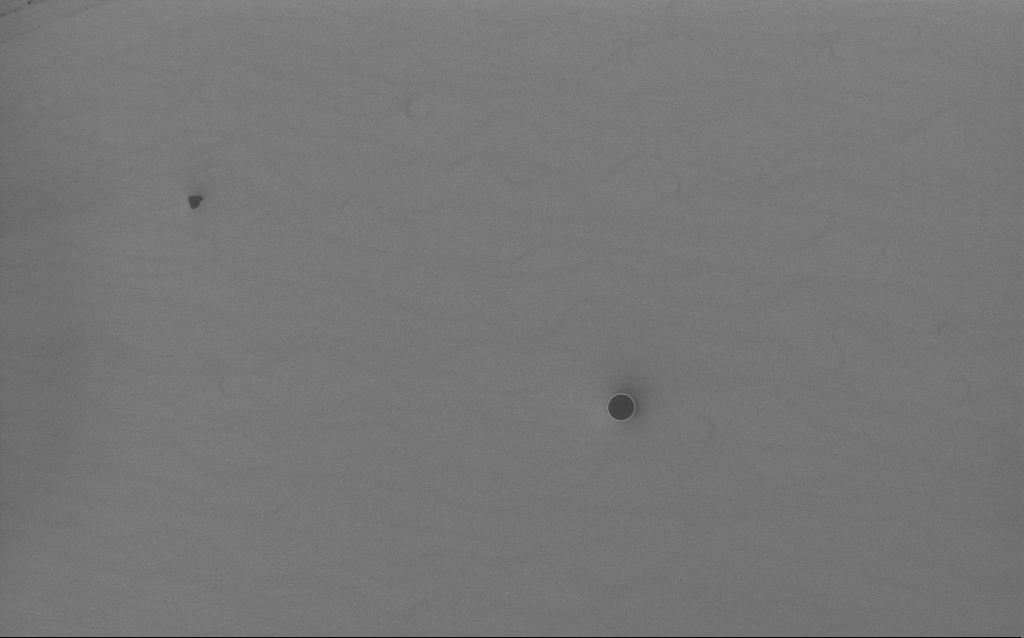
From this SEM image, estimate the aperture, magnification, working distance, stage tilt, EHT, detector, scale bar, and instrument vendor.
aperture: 30 µm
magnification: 6.59 K X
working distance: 4 mm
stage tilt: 0°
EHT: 5 kV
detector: InLens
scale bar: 10000 nm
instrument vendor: Zeiss